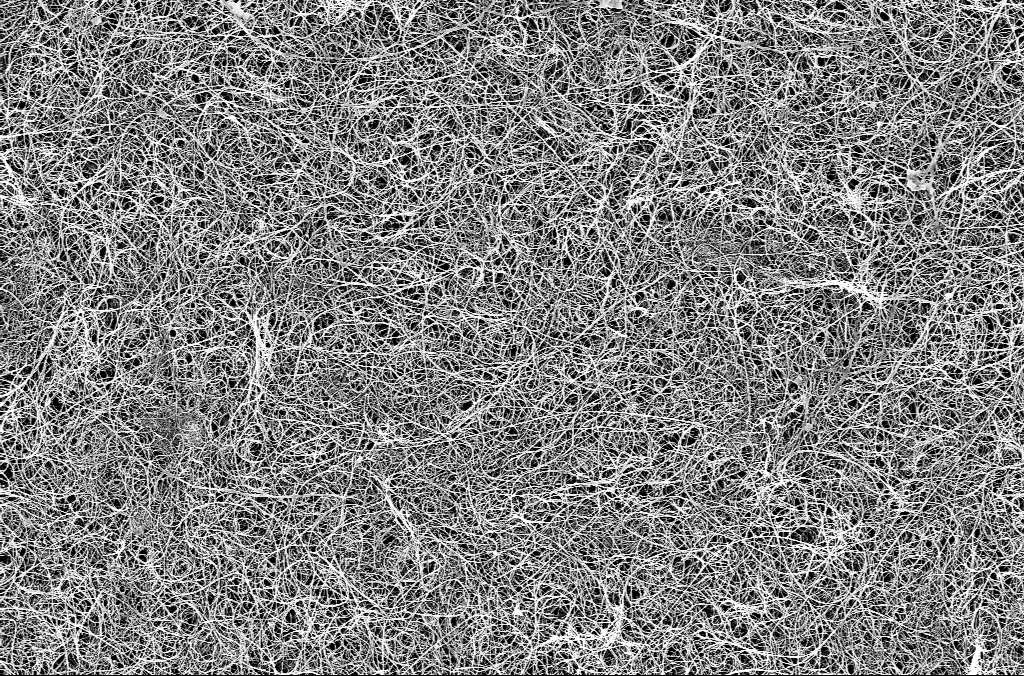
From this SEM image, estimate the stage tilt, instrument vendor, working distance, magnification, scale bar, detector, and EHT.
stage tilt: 0°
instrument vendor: Zeiss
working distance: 3 mm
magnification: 10 K X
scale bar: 2000 nm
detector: InLens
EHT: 5 kV